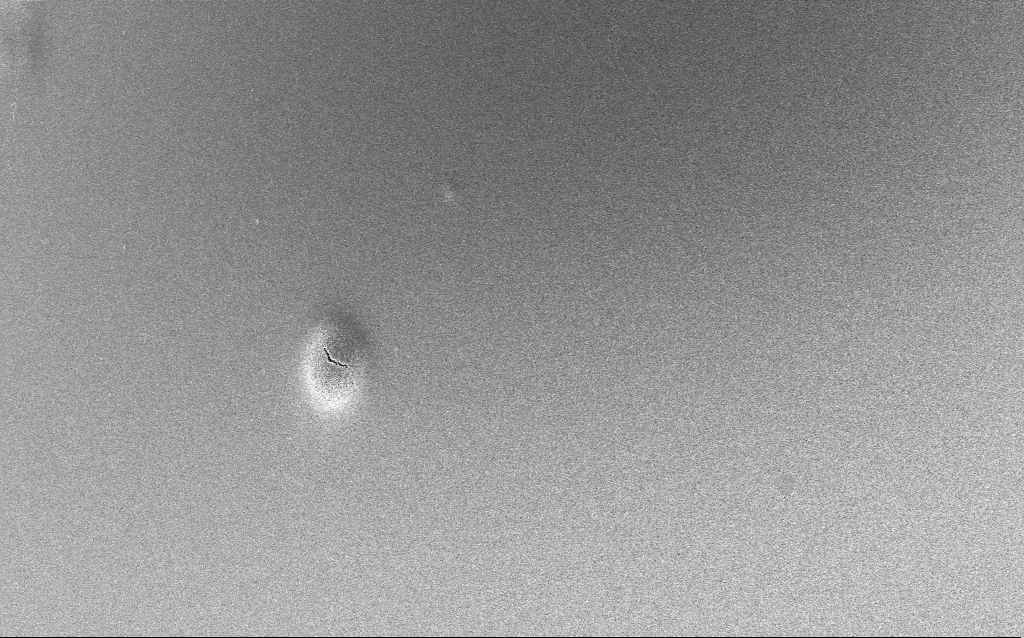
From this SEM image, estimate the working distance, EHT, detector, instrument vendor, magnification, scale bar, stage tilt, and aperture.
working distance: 2.6 mm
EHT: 5 kV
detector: InLens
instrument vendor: Zeiss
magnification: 1.69 K X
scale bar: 10000 nm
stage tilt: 0°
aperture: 30 µm